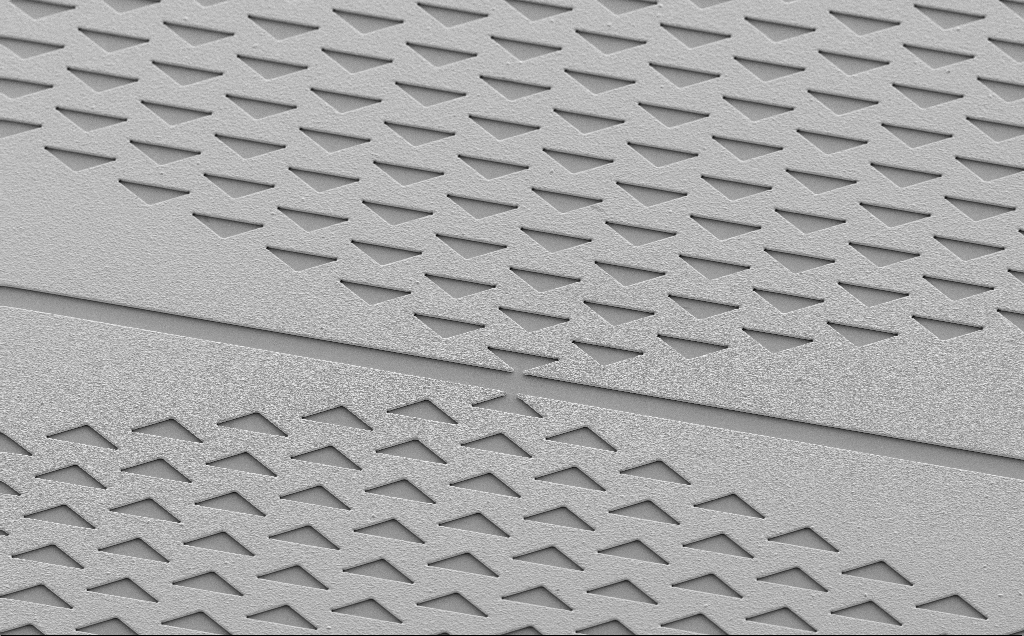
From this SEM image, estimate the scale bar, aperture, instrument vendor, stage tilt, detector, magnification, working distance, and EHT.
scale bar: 20000 nm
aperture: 30 µm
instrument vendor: Zeiss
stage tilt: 35°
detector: SE2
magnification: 0.765 K X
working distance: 13 mm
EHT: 5 kV